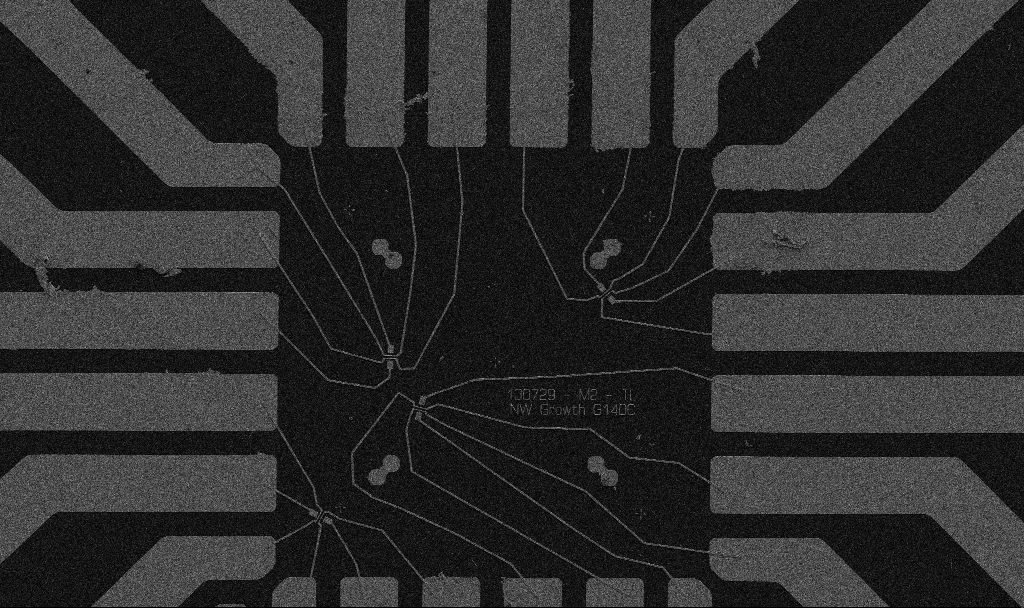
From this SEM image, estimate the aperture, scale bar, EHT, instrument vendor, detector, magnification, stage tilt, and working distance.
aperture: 30 µm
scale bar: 20000 nm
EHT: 5 kV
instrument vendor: Zeiss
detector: SE2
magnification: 1 K X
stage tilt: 0°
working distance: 10.7 mm